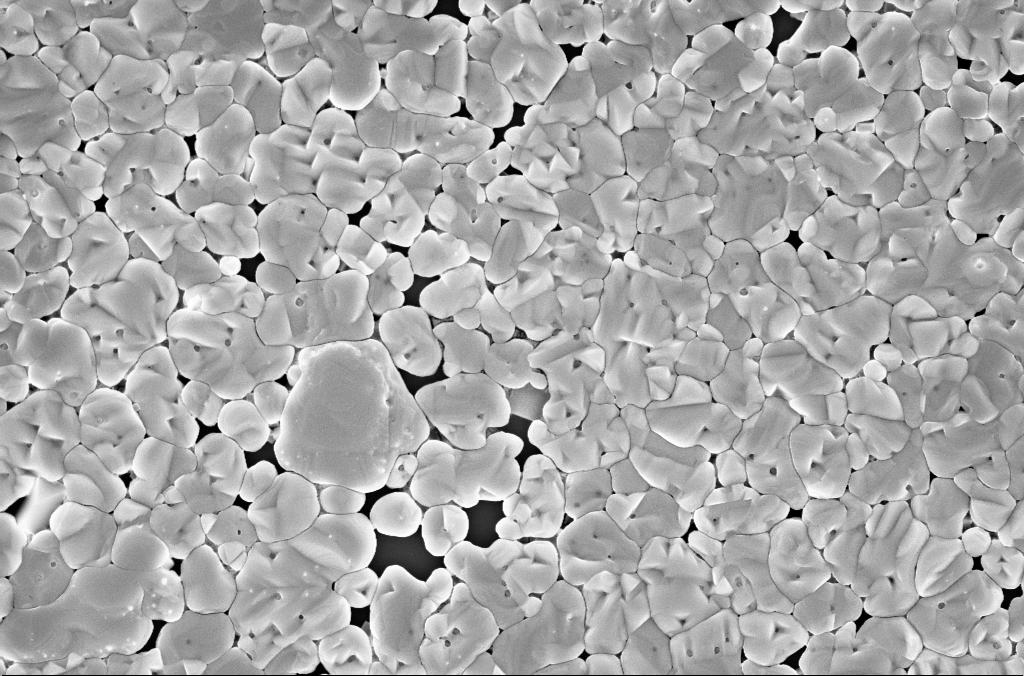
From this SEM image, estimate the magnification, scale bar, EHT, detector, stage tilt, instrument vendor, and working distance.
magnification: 30 K X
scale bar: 2000 nm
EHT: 5 kV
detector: InLens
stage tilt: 0°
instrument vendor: Zeiss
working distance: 3 mm